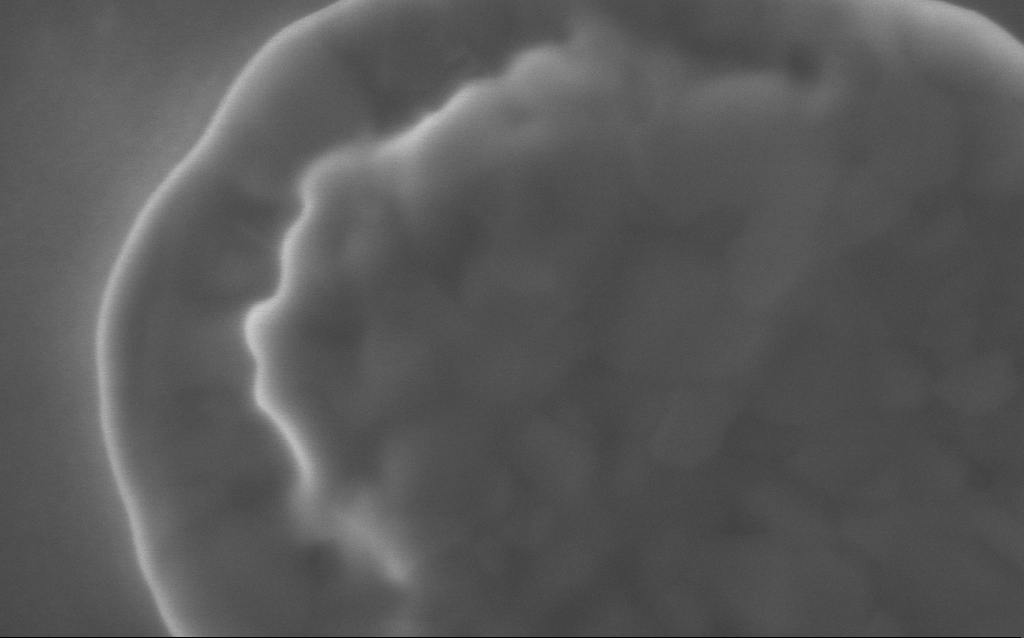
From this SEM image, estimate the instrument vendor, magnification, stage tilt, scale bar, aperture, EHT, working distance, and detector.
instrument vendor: Zeiss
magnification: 217.21 K X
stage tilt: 0°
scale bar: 200 nm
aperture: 30 µm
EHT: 5 kV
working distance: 3 mm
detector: InLens